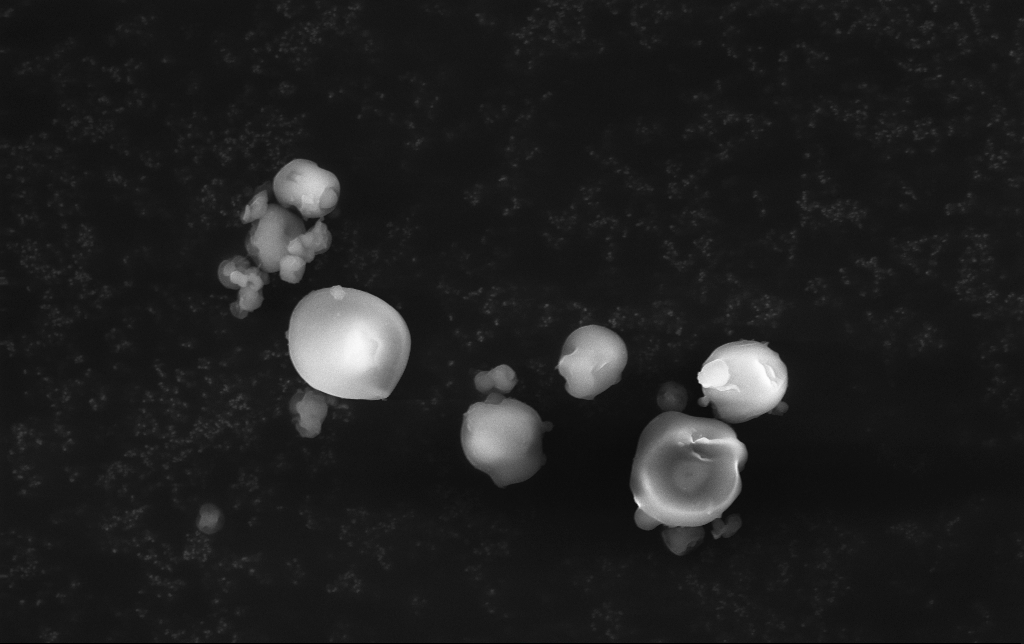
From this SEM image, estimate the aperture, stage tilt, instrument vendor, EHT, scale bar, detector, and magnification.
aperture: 30 µm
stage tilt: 0°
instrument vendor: Zeiss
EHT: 15 kV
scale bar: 2000 nm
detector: InLens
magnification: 8.08 K X